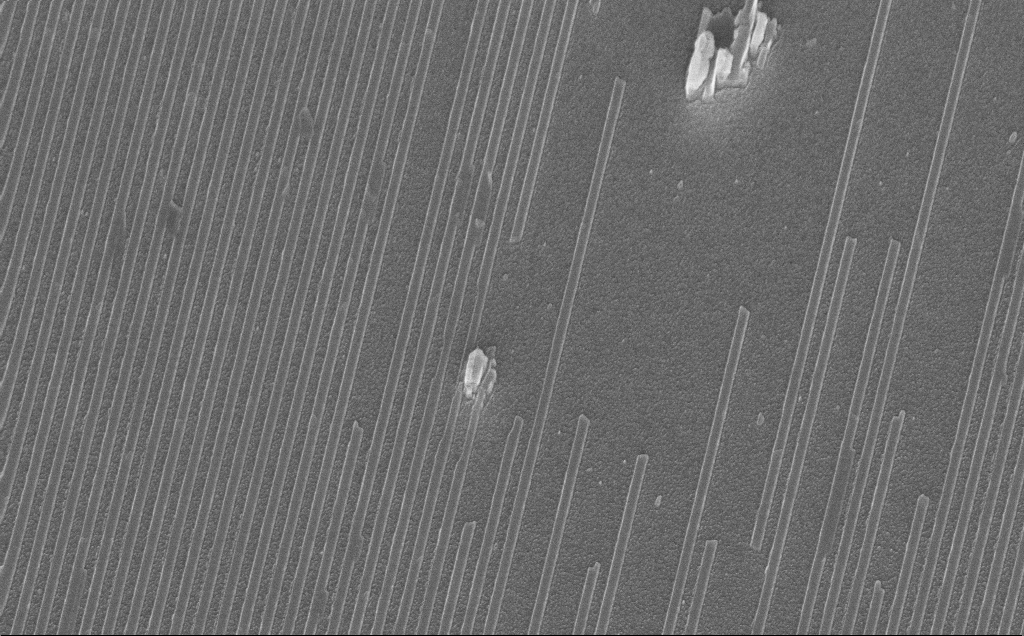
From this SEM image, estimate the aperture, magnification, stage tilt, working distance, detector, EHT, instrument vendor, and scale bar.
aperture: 30 µm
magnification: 16.01 K X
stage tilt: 38°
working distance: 6 mm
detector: InLens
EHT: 10 kV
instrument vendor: Zeiss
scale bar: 2000 nm